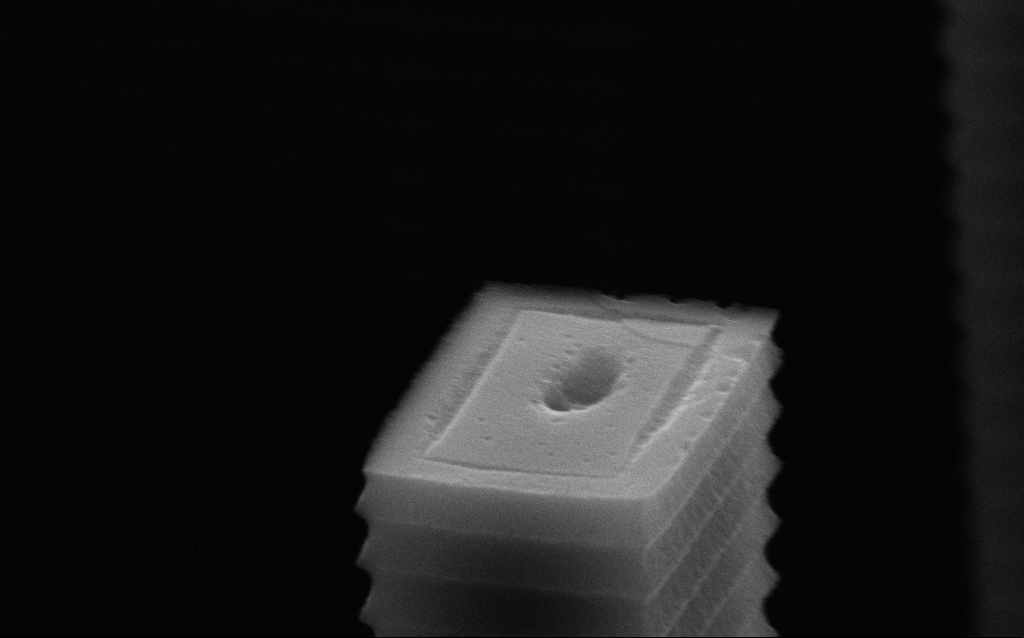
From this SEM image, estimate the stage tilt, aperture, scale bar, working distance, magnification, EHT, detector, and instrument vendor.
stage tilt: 70°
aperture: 30 µm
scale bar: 1000 nm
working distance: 7.2 mm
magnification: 63.87 K X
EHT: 10 kV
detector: SE2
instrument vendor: Zeiss